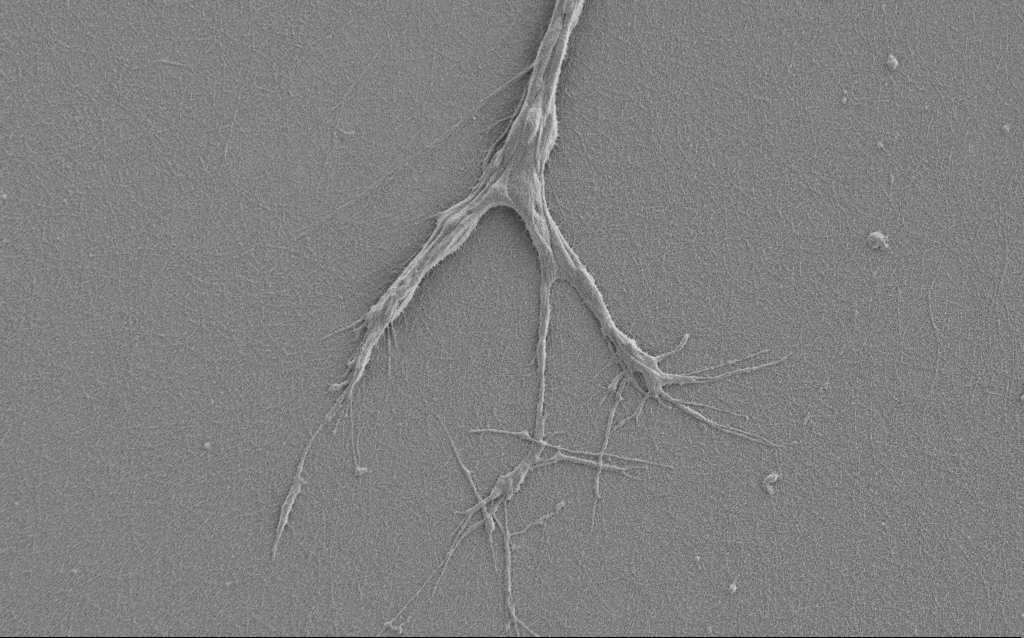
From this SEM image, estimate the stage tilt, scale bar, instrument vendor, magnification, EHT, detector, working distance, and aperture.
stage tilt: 0°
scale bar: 10000 nm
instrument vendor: Zeiss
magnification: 6 K X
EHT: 1 kV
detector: SE2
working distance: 6 mm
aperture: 30 µm